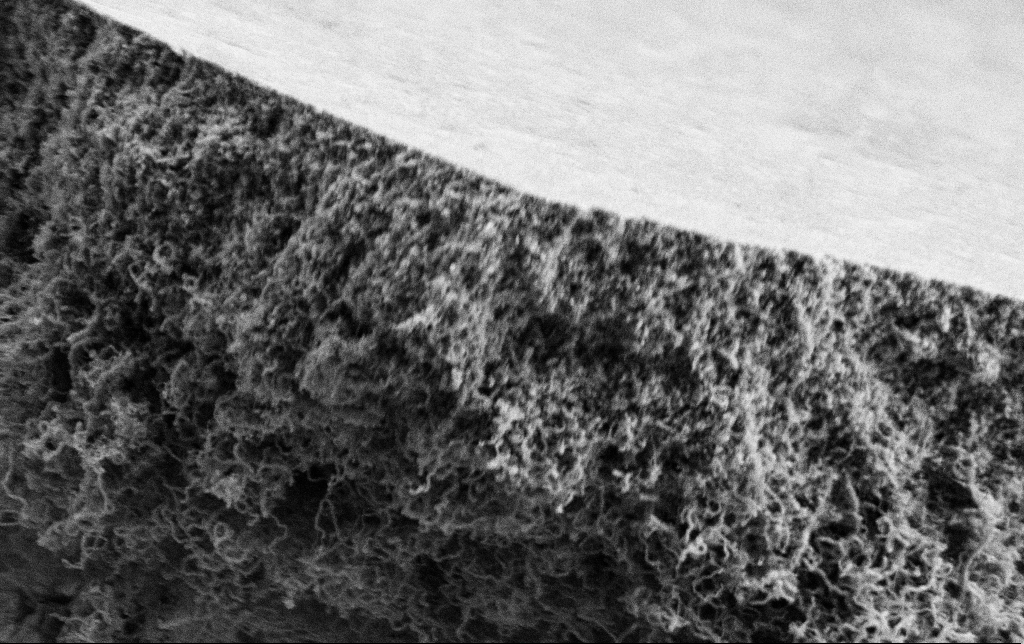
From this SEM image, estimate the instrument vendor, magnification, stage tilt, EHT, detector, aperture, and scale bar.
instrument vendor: Zeiss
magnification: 25 K X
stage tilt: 0°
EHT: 2 kV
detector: SE2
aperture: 30 µm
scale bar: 2000 nm